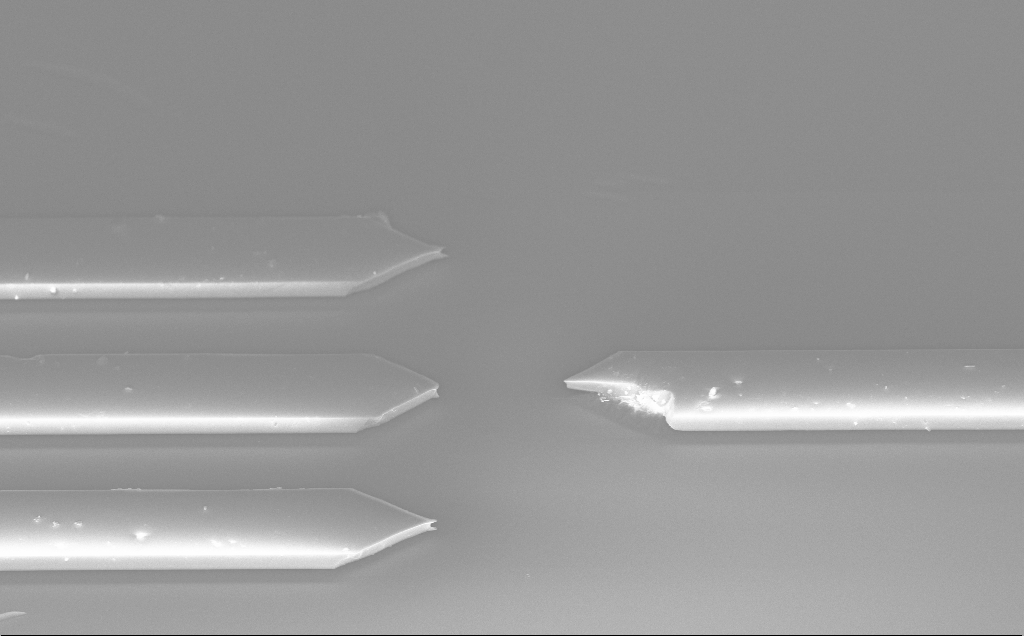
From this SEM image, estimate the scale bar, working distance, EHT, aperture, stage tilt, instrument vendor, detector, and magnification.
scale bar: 10000 nm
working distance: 10 mm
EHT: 10 kV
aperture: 30 µm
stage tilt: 50°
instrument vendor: Zeiss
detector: InLens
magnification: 1.94 K X